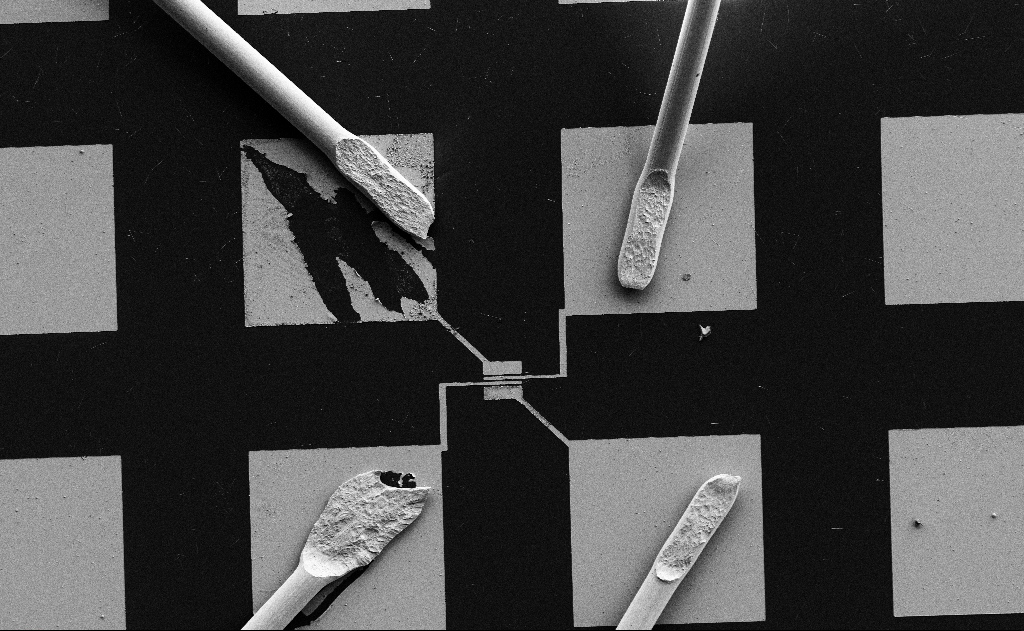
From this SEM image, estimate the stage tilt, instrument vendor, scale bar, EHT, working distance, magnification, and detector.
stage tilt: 0°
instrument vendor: Zeiss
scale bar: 100000 nm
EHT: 5 kV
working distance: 15 mm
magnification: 0.48 K X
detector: SE2